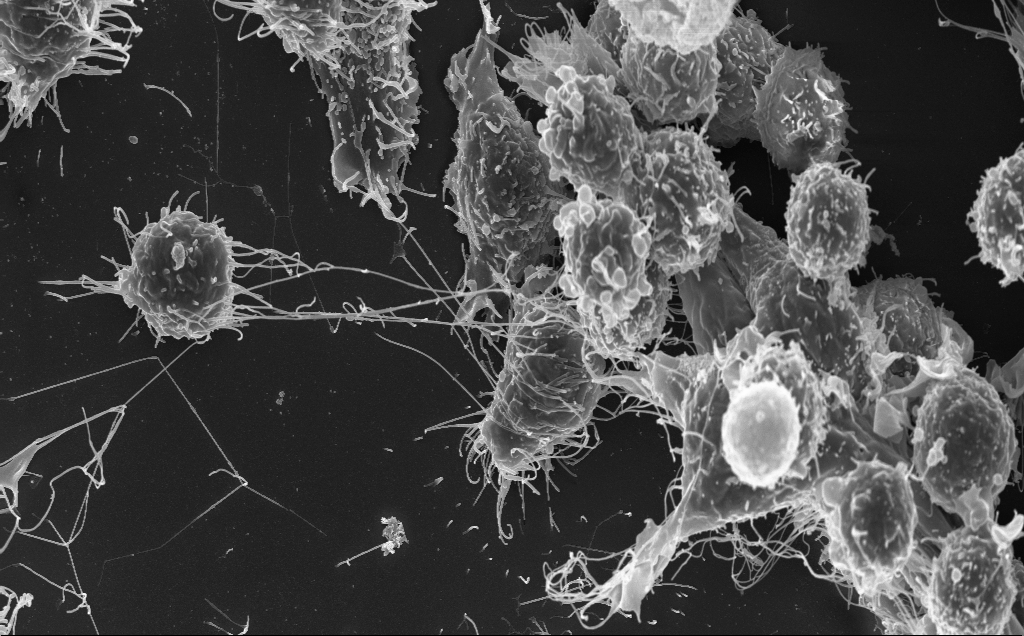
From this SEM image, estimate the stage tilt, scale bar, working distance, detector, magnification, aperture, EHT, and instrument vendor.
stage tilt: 0.7°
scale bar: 10000 nm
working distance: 5 mm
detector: InLens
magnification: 4.7 K X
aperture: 30 µm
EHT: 10 kV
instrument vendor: Zeiss